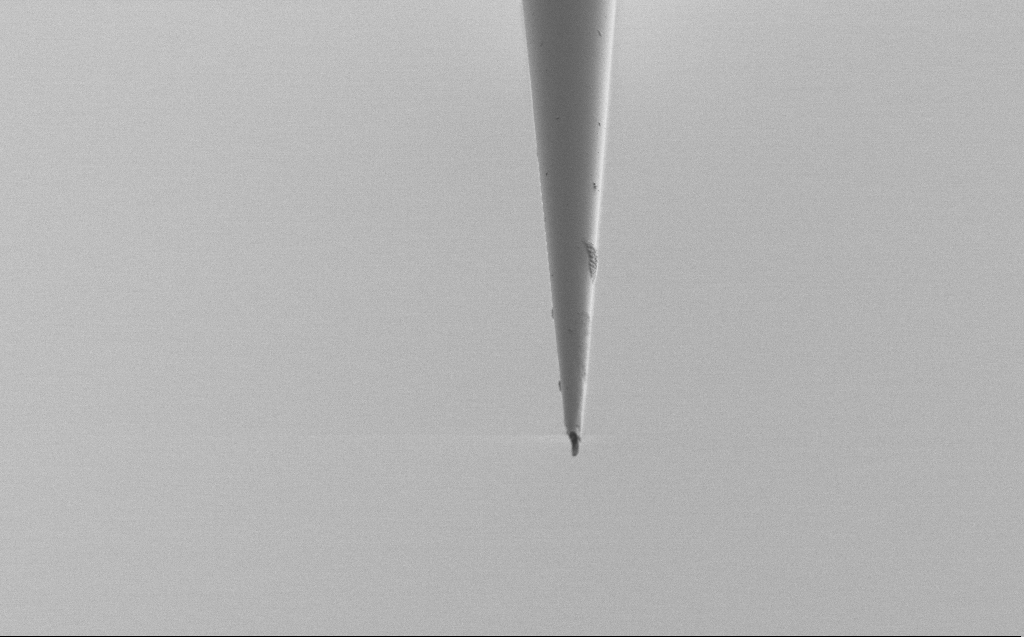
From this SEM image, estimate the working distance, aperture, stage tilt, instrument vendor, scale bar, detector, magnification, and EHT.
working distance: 5 mm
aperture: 30 µm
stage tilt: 45°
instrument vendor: Zeiss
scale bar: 10000 nm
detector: SE2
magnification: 5 K X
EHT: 1 kV